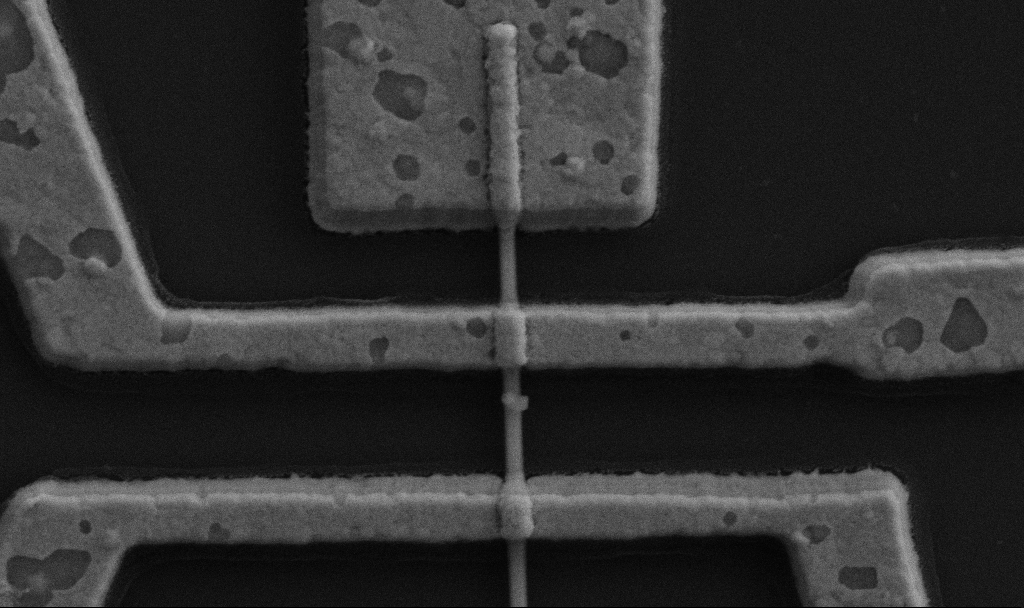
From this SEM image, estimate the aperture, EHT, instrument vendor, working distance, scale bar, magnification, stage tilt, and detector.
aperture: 30 µm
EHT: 5 kV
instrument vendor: Zeiss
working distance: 9.7 mm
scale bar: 1000 nm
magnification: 60 K X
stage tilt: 0°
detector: SE2